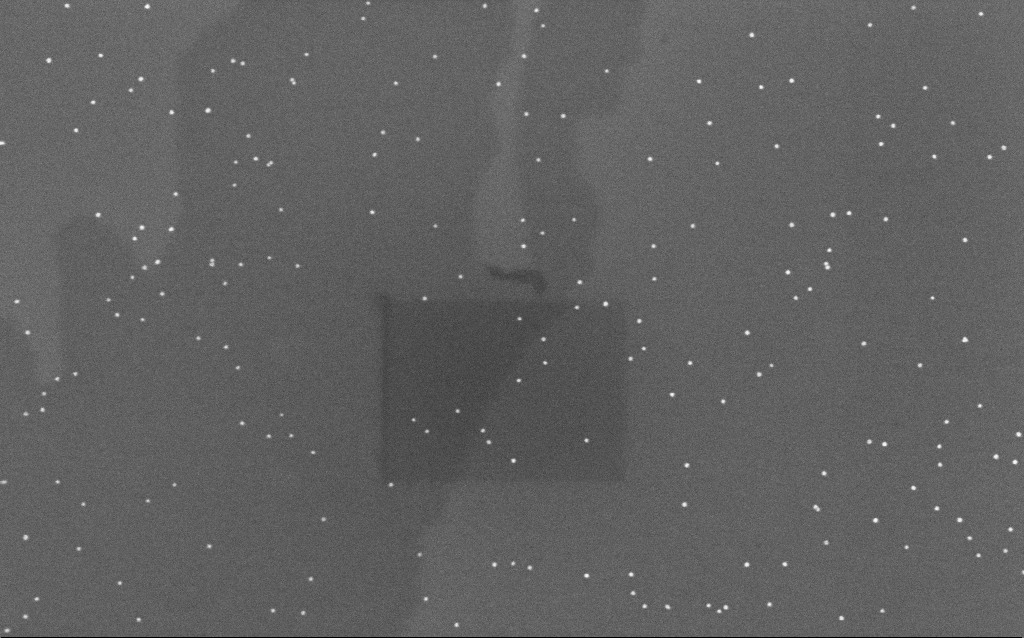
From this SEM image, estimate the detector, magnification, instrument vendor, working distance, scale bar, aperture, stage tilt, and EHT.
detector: InLens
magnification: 100 K X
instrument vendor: Zeiss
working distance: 6.6 mm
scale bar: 200 nm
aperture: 30 µm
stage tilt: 0°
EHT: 10 kV